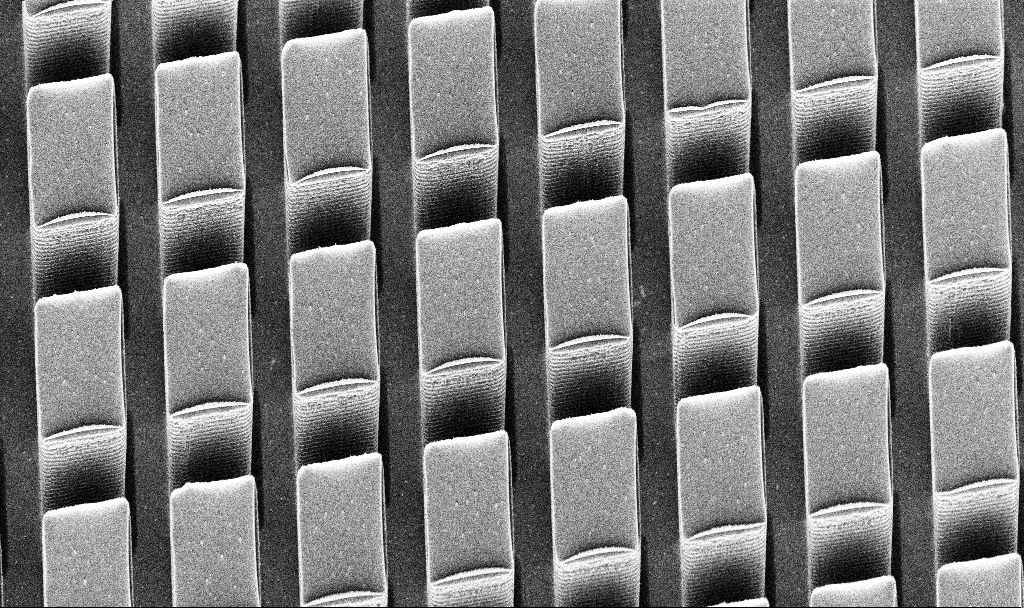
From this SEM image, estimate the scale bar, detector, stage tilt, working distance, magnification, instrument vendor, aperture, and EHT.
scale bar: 10000 nm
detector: InLens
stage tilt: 30°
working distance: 9.4 mm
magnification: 3.88 K X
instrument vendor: Zeiss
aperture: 30 µm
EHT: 5 kV